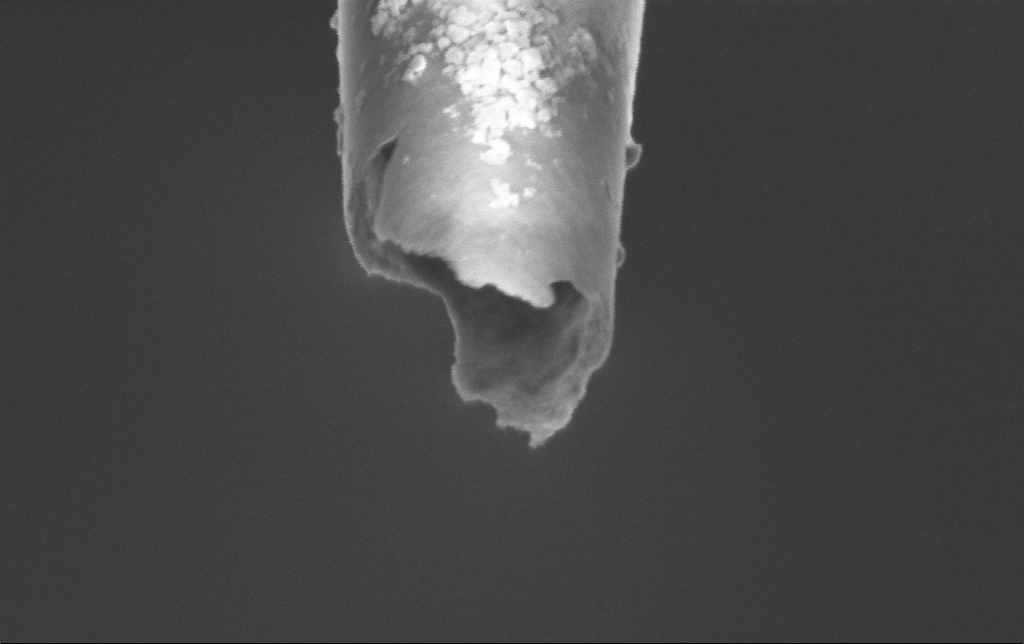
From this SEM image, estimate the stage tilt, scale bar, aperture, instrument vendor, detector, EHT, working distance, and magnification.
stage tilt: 45°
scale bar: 200 nm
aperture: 30 µm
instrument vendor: Zeiss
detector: InLens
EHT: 2 kV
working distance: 7.4 mm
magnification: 100 K X